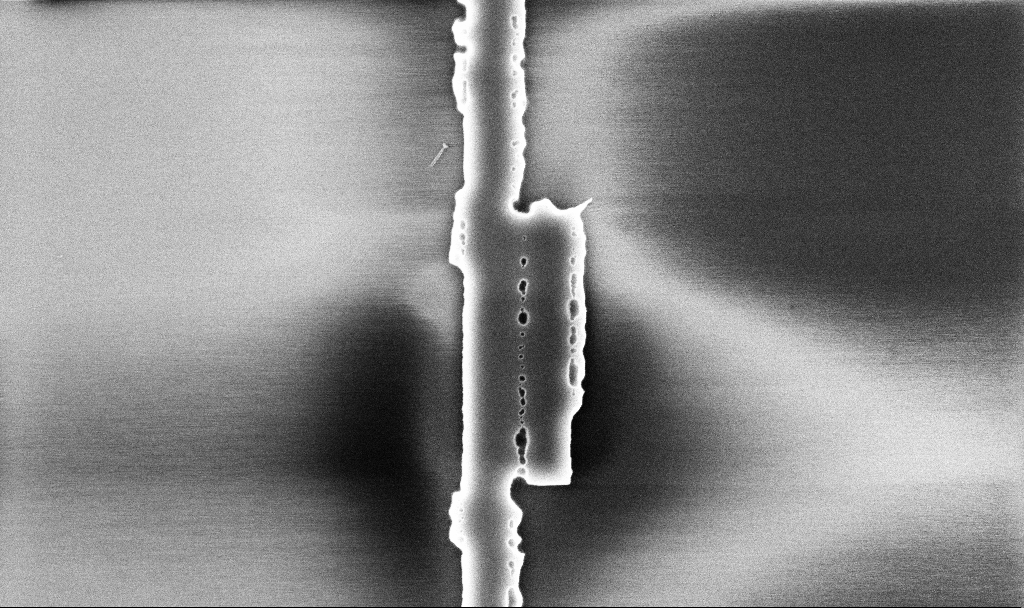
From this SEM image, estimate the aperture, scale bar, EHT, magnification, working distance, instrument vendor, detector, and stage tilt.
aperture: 30 µm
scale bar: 2000 nm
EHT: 5 kV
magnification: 34.16 K X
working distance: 10.1 mm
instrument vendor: Zeiss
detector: InLens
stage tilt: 0°